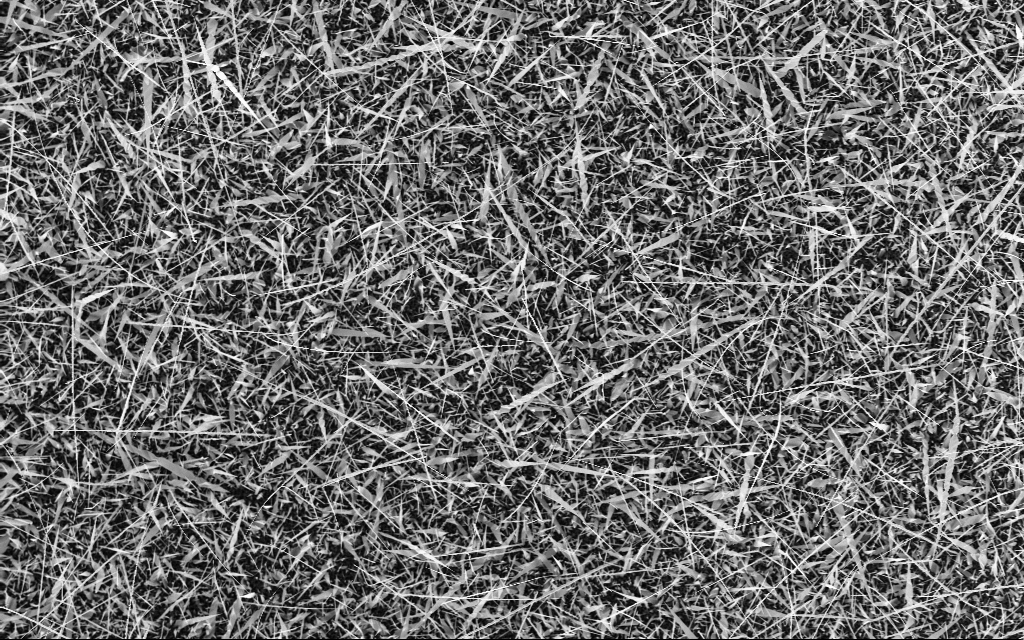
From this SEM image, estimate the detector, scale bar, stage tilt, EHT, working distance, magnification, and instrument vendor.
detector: InLens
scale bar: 10000 nm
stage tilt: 0°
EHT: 10 kV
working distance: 6 mm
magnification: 3 K X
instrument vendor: Zeiss